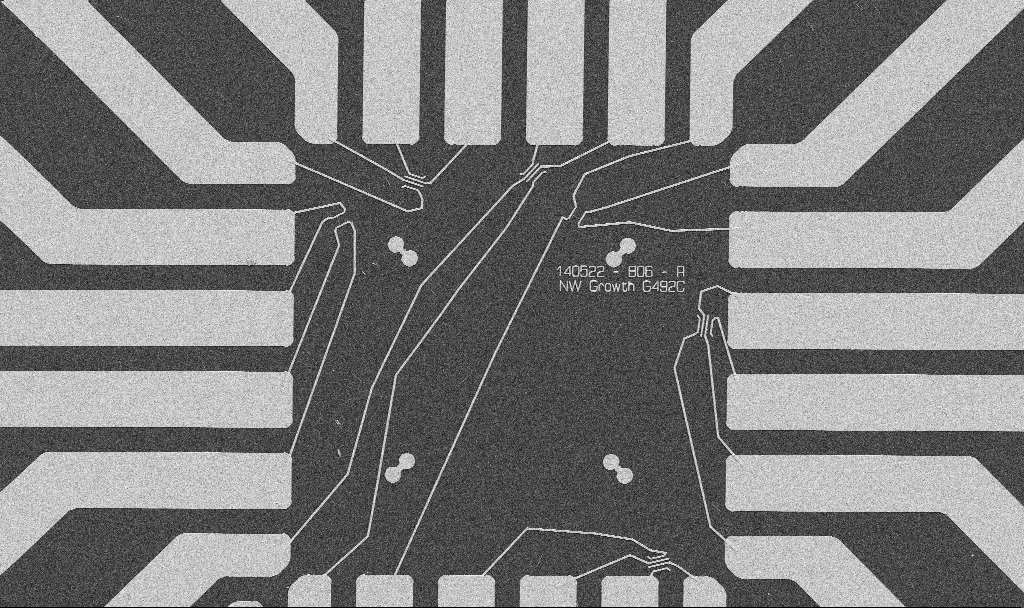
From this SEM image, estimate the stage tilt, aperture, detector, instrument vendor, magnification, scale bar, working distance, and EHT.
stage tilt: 0°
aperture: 30 µm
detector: SE2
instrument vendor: Zeiss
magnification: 1 K X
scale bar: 20000 nm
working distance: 10.7 mm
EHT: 5 kV